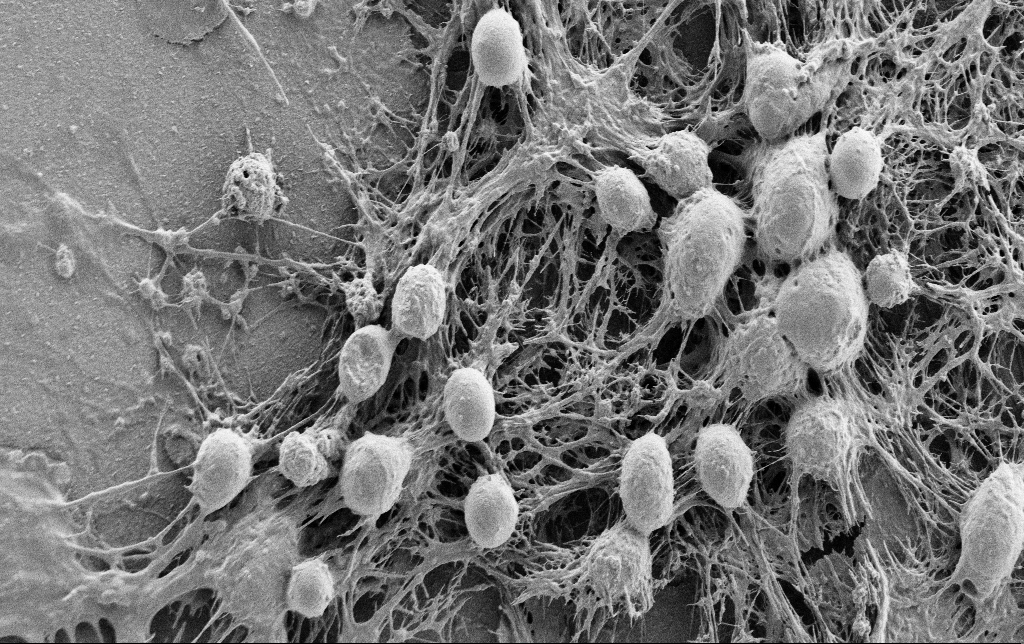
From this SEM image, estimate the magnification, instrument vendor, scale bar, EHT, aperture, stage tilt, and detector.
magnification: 5 K X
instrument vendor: Zeiss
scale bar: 10000 nm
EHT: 0.9 kV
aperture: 30 µm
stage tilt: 0°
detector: SE2